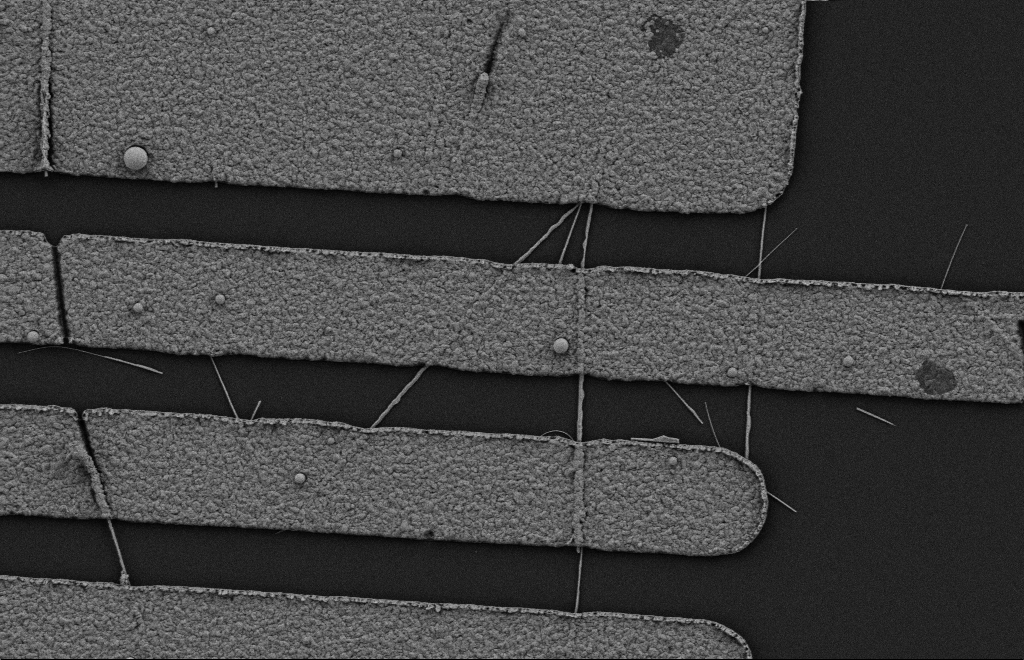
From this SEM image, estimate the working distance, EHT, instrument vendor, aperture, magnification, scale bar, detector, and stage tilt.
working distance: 10 mm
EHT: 2 kV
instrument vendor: Zeiss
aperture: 20 µm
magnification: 15.75 K X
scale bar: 2000 nm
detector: SE2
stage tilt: -0.3°